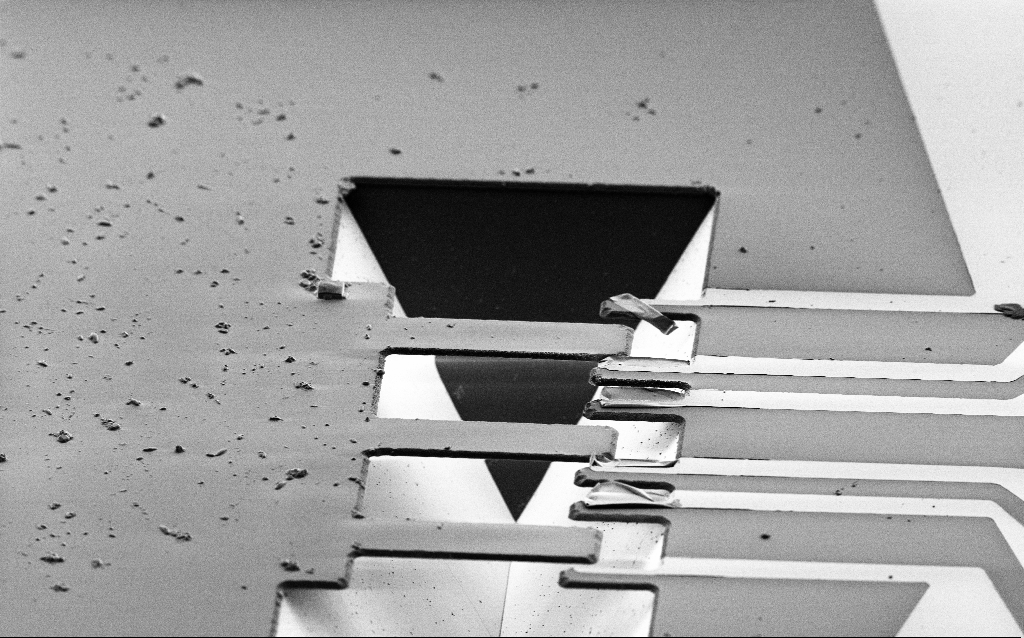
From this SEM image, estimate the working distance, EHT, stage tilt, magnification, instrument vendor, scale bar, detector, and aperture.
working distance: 9.2 mm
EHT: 2 kV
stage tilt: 59°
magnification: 1.19 K X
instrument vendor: Zeiss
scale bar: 20000 nm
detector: SE2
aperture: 30 µm